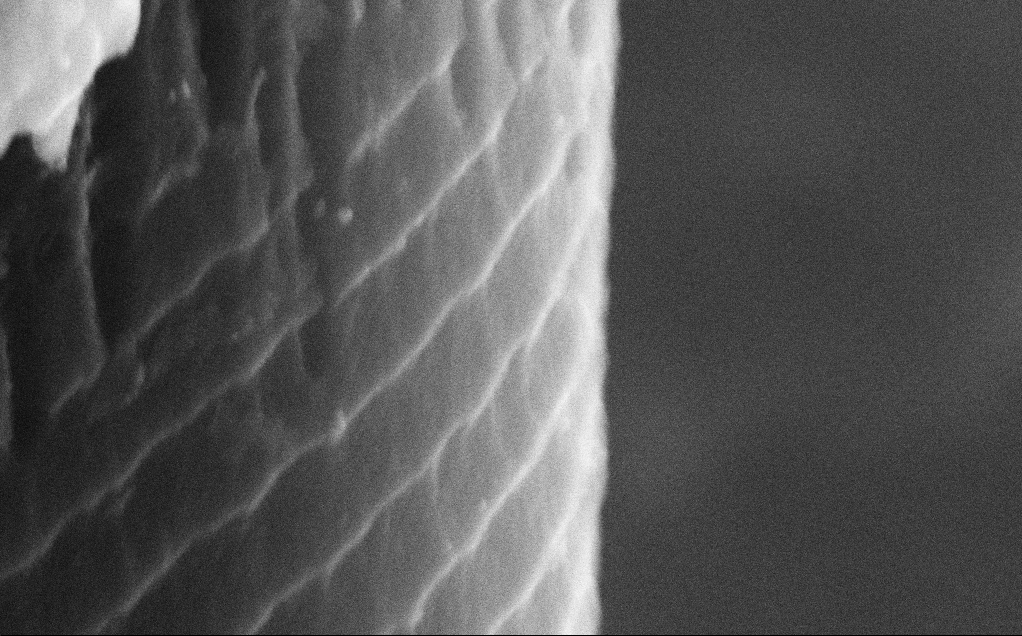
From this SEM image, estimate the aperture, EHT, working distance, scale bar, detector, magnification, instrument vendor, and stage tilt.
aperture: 30 µm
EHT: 15 kV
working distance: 10 mm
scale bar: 200 nm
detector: SE2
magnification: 125.8 K X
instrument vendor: Zeiss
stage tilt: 50°